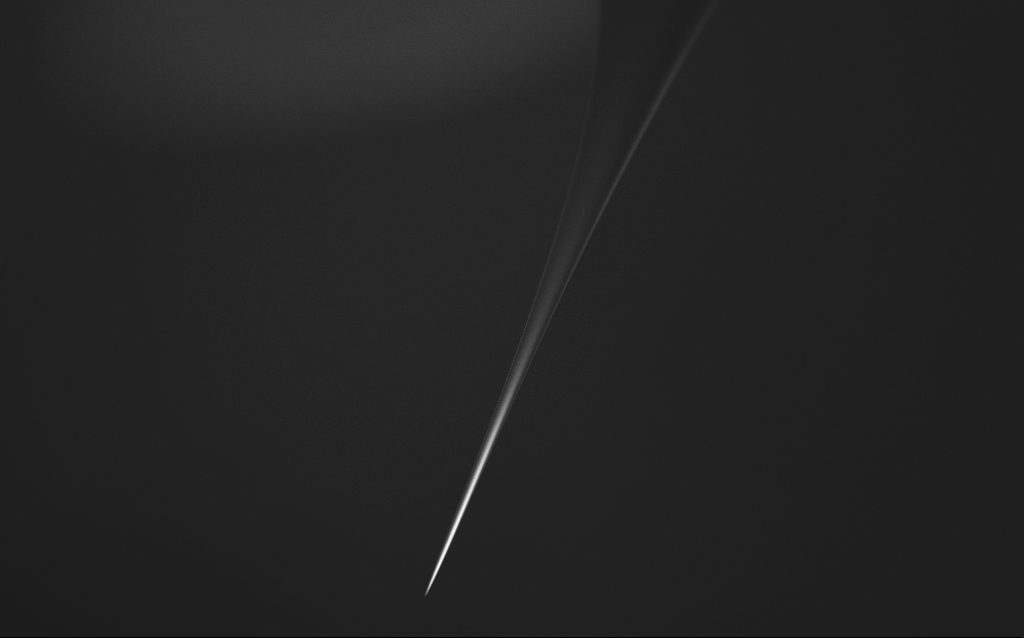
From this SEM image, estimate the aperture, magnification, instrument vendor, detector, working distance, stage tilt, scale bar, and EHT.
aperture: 30 µm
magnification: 0.1 K X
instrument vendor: Zeiss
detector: InLens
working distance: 5 mm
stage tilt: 45°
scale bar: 200000 nm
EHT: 2.5 kV